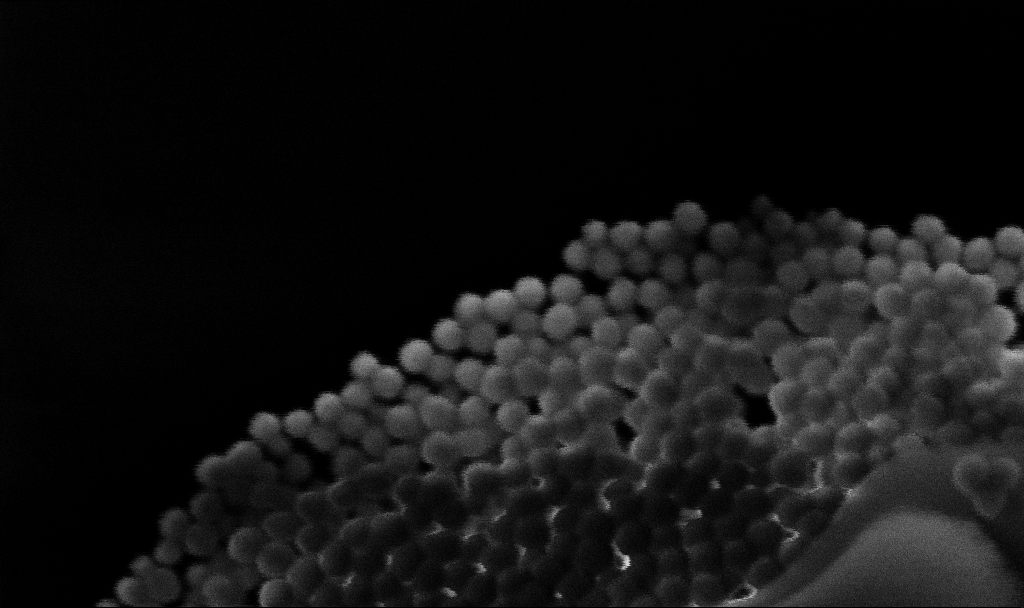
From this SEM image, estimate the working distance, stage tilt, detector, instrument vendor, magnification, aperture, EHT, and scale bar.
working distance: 3.1 mm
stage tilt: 0°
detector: InLens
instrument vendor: Zeiss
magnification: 618.82 K X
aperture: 30 µm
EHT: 4 kV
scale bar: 100 nm